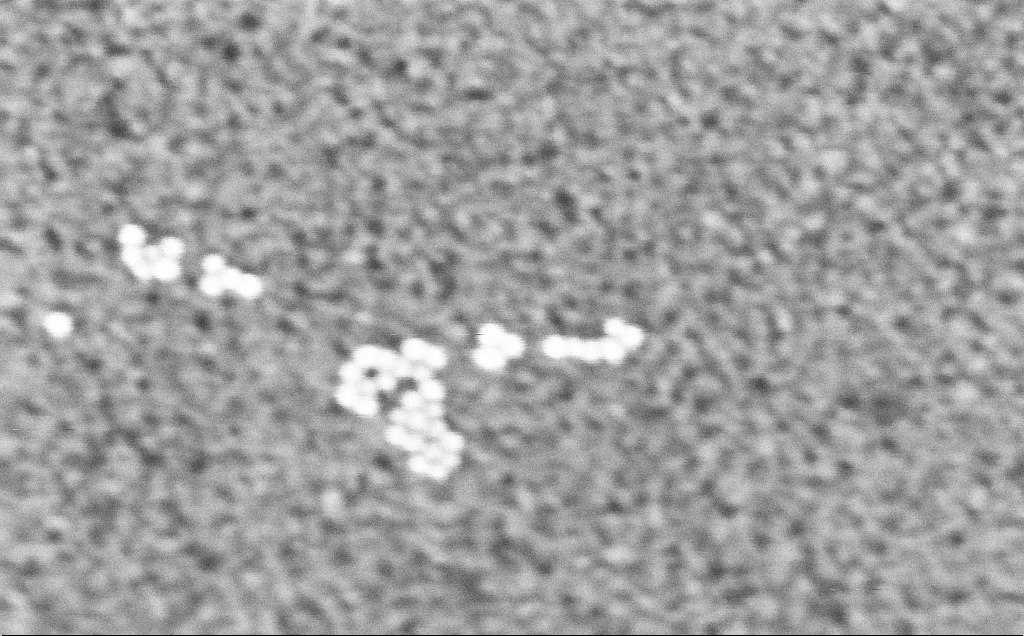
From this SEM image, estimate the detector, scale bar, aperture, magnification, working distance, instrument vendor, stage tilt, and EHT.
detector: InLens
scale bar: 100 nm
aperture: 30 µm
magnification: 466.24 K X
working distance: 3.2 mm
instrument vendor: Zeiss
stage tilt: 0°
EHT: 3 kV